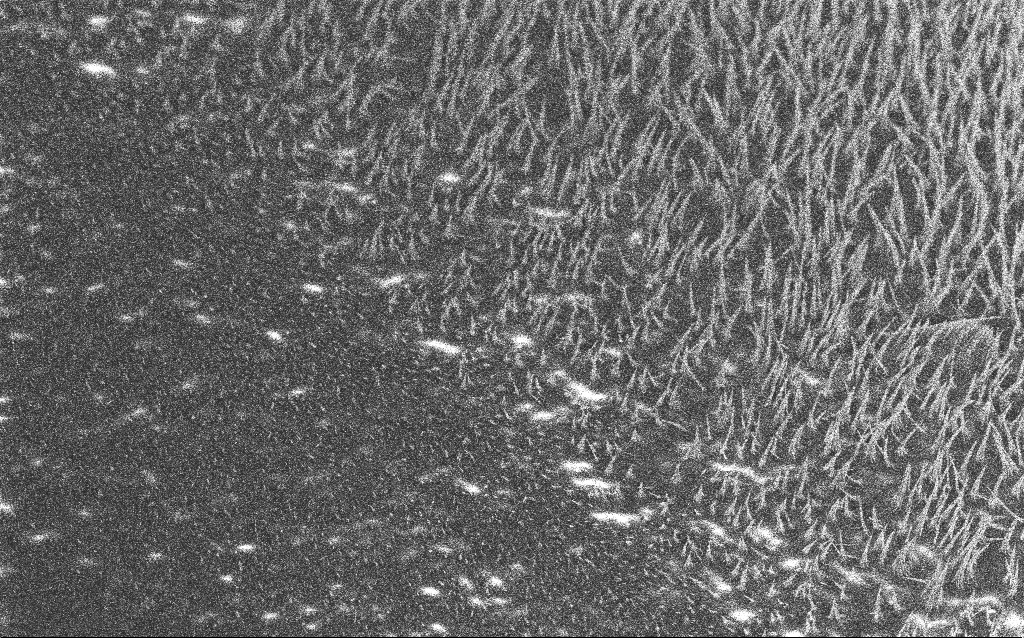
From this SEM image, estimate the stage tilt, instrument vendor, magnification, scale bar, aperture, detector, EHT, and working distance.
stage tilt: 40°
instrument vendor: Zeiss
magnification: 5.29 K X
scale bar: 10000 nm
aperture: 30 µm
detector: InLens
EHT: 5 kV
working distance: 6.3 mm